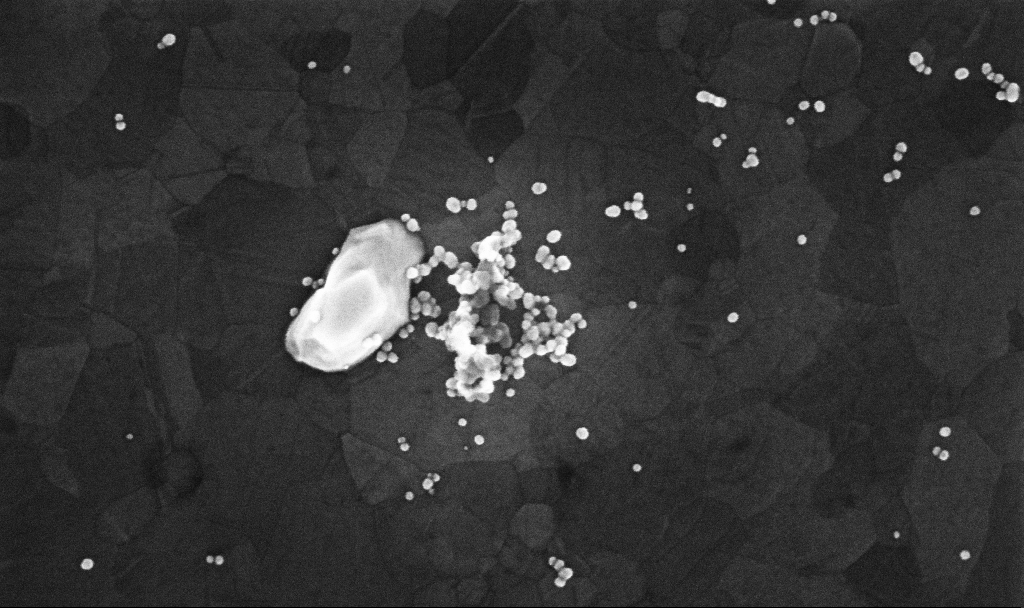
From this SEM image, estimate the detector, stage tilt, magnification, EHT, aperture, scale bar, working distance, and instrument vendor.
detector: InLens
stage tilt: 0°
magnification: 179.42 K X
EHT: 10 kV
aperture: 30 µm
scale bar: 200 nm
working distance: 3.4 mm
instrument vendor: Zeiss